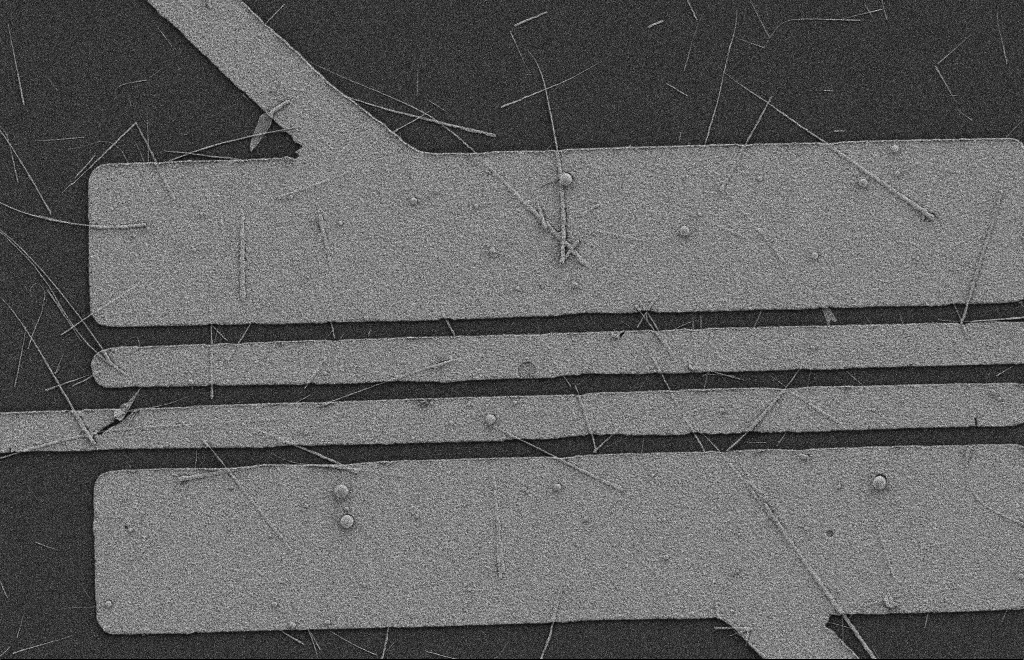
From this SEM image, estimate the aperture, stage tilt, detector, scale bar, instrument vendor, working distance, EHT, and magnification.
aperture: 20 µm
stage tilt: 0°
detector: SE2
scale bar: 2000 nm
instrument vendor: Zeiss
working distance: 8 mm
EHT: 2 kV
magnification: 5.63 K X